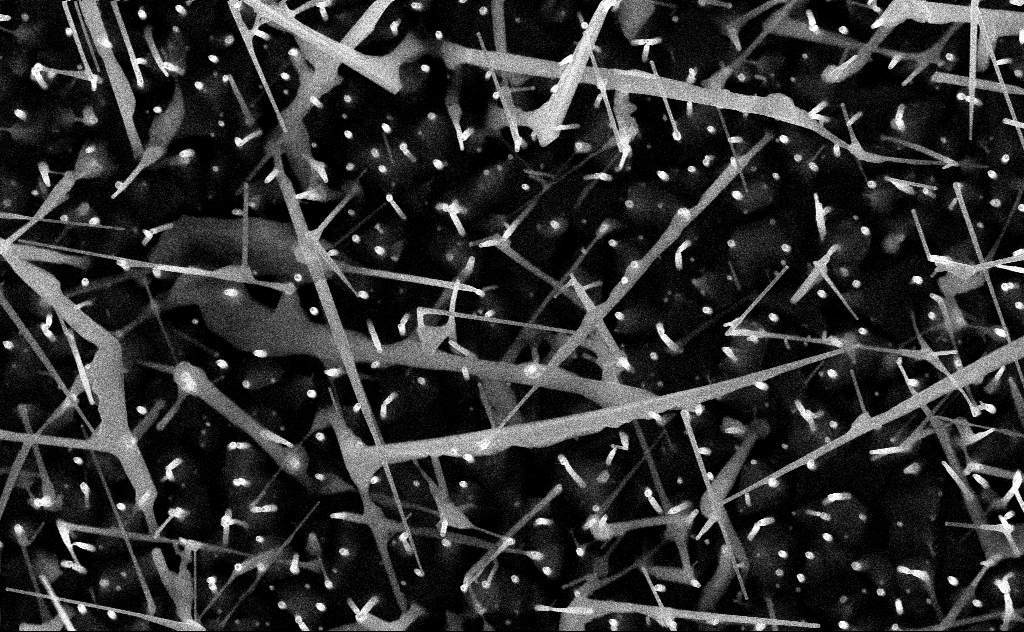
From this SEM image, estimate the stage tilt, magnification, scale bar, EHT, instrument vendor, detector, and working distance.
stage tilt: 0°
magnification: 40 K X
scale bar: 1000 nm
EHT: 10 kV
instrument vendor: Zeiss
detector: InLens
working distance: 7 mm